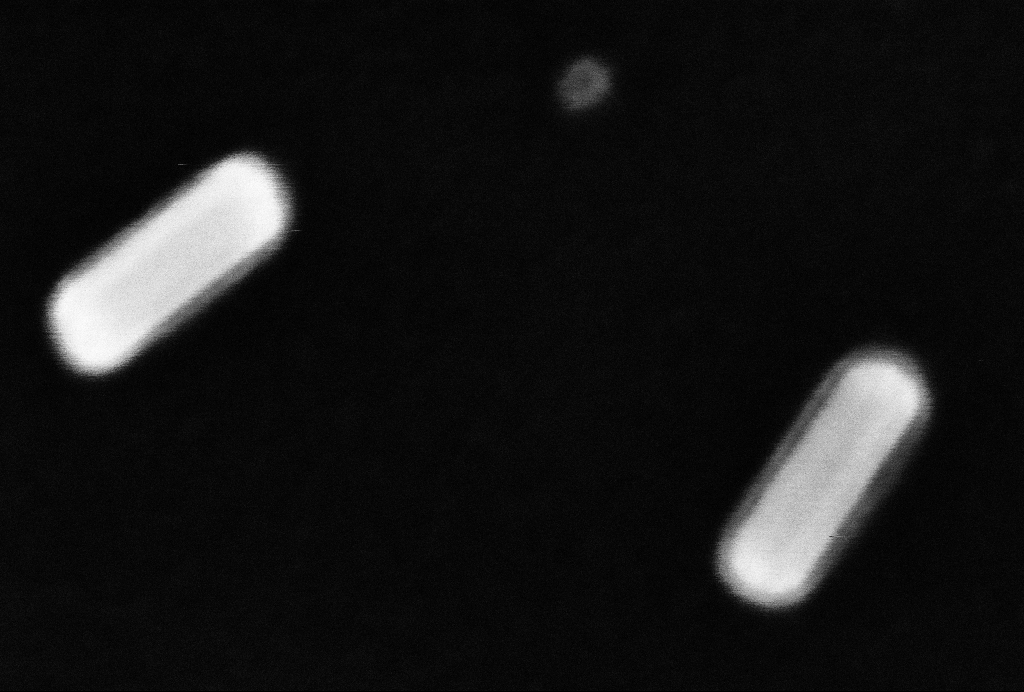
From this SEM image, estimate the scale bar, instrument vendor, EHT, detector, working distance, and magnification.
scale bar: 20 nm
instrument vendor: Zeiss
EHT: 10 kV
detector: InLens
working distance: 3.2 mm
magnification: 1354.79 K X